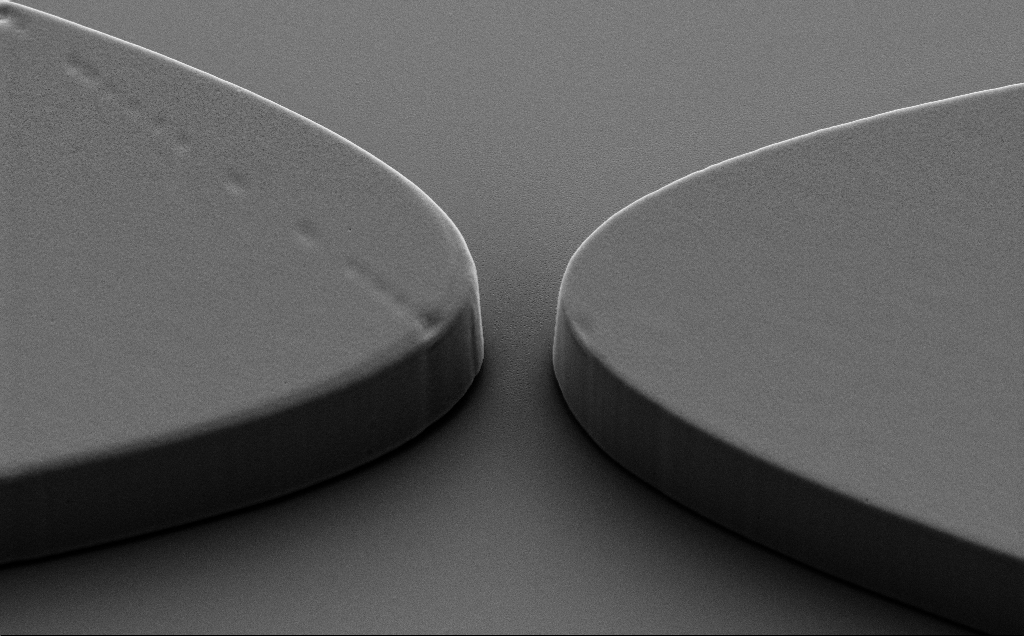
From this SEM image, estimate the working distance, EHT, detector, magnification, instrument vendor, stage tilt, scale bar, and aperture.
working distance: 8 mm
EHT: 5 kV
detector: SE2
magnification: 5 K X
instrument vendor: Zeiss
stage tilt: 40°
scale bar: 10000 nm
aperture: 30 µm